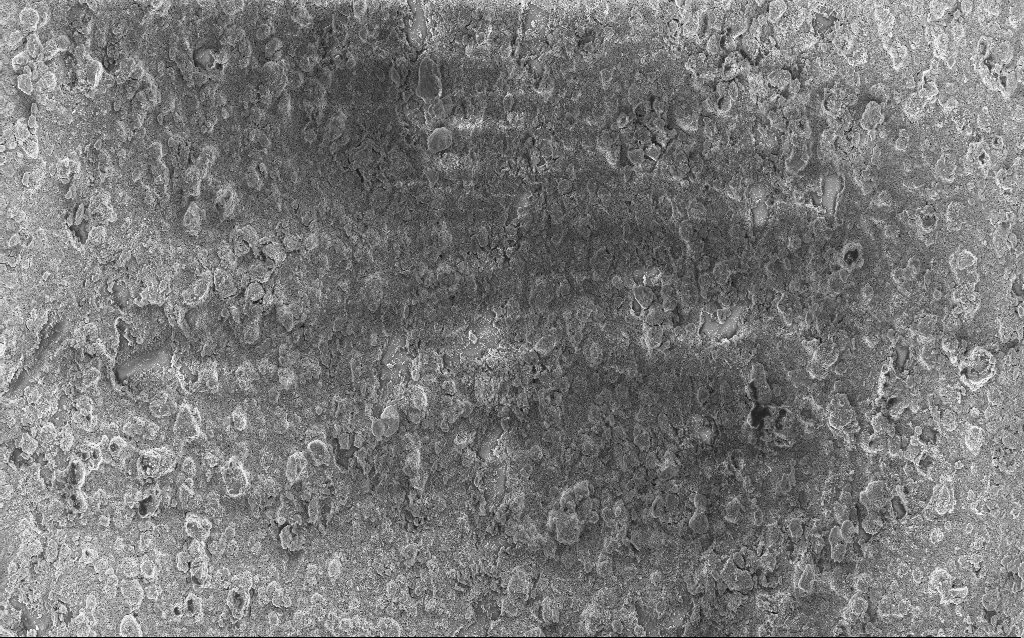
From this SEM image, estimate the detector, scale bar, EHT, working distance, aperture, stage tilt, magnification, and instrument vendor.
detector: InLens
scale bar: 20000 nm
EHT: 5 kV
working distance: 2.5 mm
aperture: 30 µm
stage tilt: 0°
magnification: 0.792 K X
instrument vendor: Zeiss